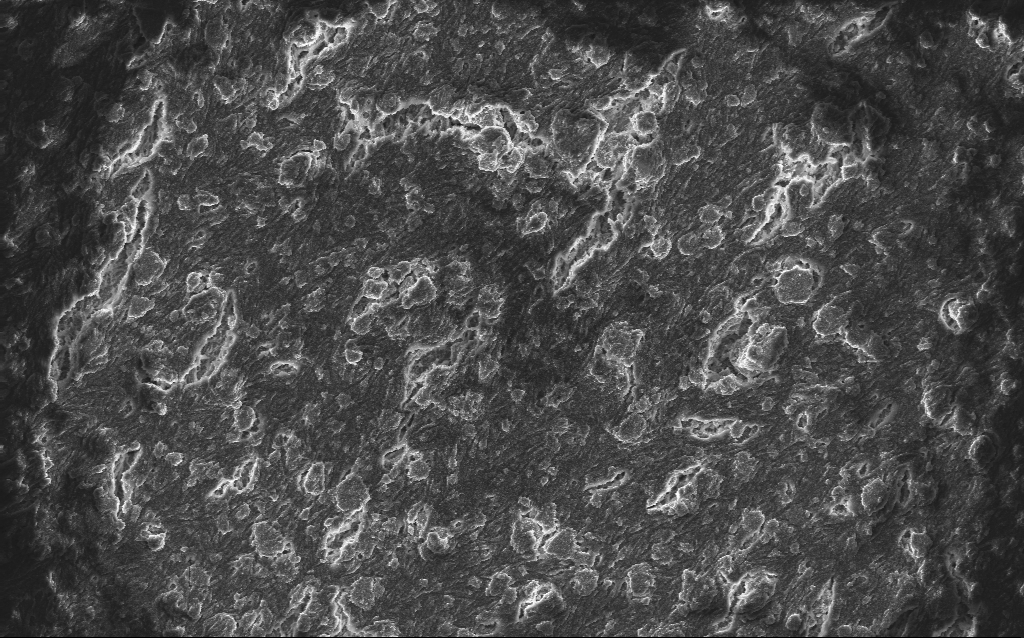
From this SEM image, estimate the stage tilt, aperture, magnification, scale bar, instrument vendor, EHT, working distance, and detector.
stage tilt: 0°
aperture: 30 µm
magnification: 1.69 K X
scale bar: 10000 nm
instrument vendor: Zeiss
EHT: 10 kV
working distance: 2.6 mm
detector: InLens